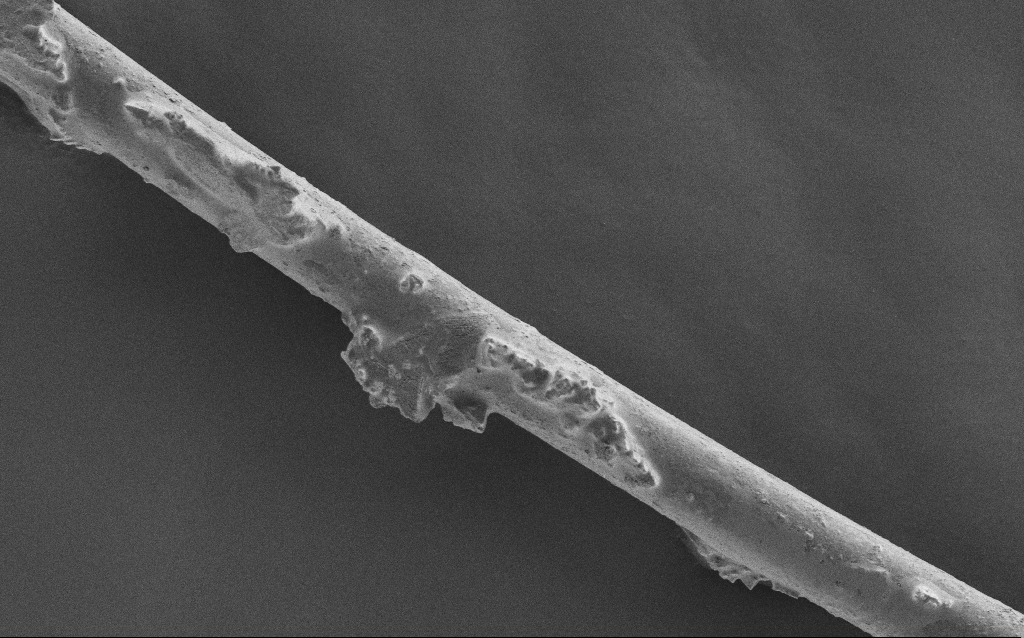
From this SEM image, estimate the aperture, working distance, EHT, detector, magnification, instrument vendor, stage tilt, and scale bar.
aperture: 30 µm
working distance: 5 mm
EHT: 1 kV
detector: SE2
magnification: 1.45 K X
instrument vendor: Zeiss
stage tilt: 0°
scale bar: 20000 nm